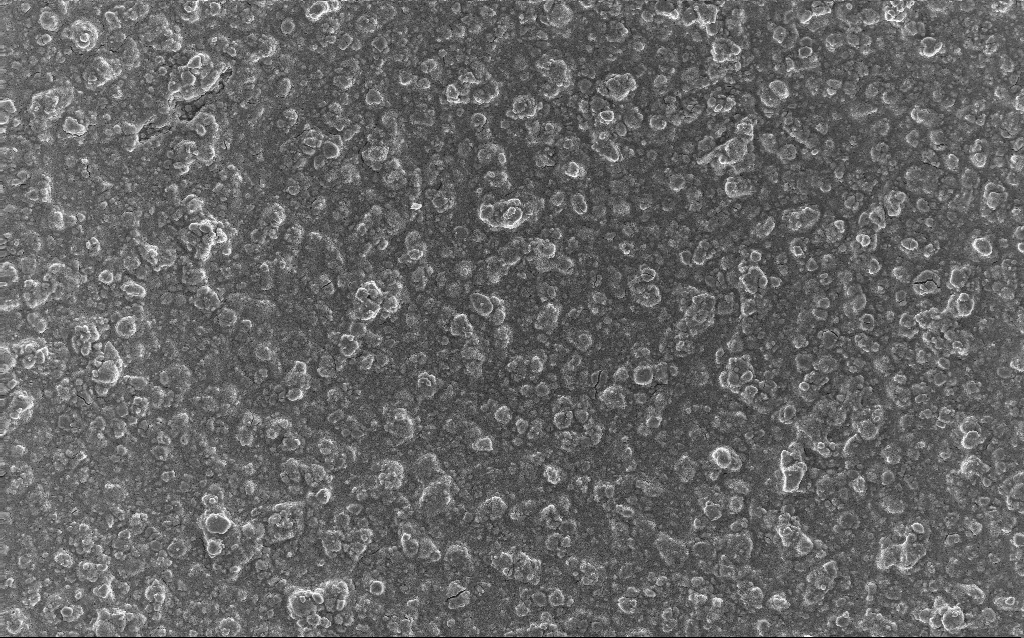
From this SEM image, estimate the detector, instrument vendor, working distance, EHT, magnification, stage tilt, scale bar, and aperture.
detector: InLens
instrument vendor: Zeiss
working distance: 2.8 mm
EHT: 10 kV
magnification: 0.77 K X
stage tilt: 0°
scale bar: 20000 nm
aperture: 30 µm